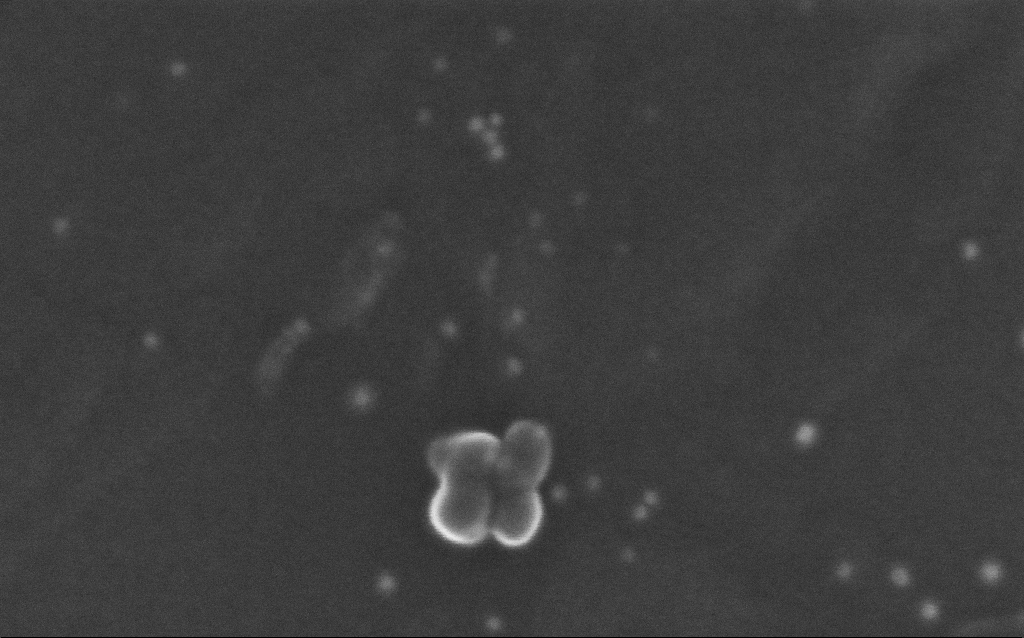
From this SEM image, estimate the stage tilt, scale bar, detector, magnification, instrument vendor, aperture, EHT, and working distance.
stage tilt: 0°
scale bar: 100 nm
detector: InLens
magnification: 420.19 K X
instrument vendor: Zeiss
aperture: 30 µm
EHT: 10 kV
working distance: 6.6 mm